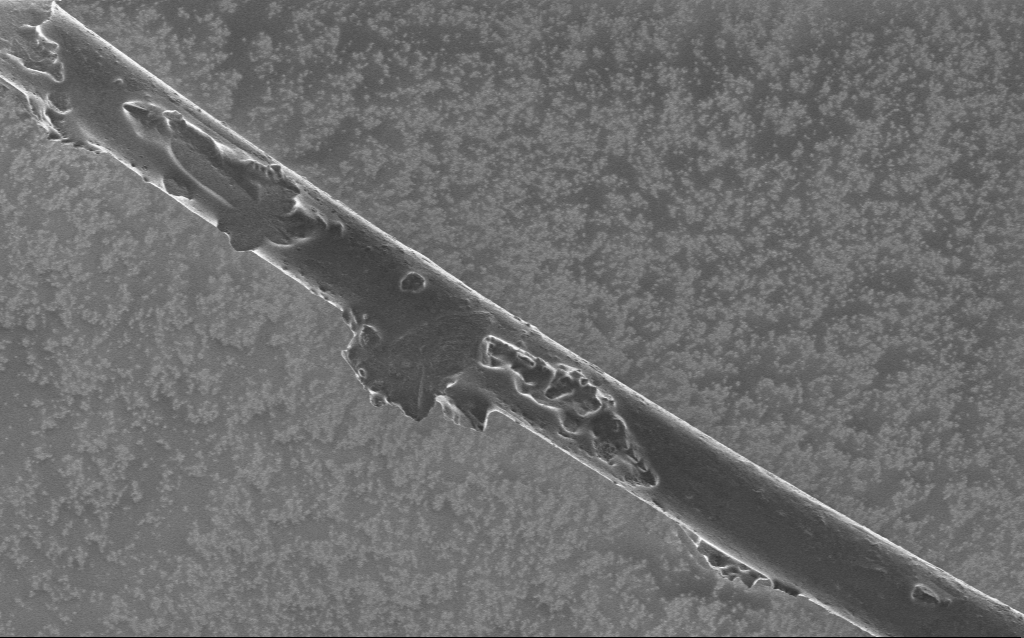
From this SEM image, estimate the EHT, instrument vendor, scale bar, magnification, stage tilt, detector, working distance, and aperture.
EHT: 1 kV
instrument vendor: Zeiss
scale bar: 20000 nm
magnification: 1.45 K X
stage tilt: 0°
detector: InLens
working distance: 5 mm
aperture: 30 µm